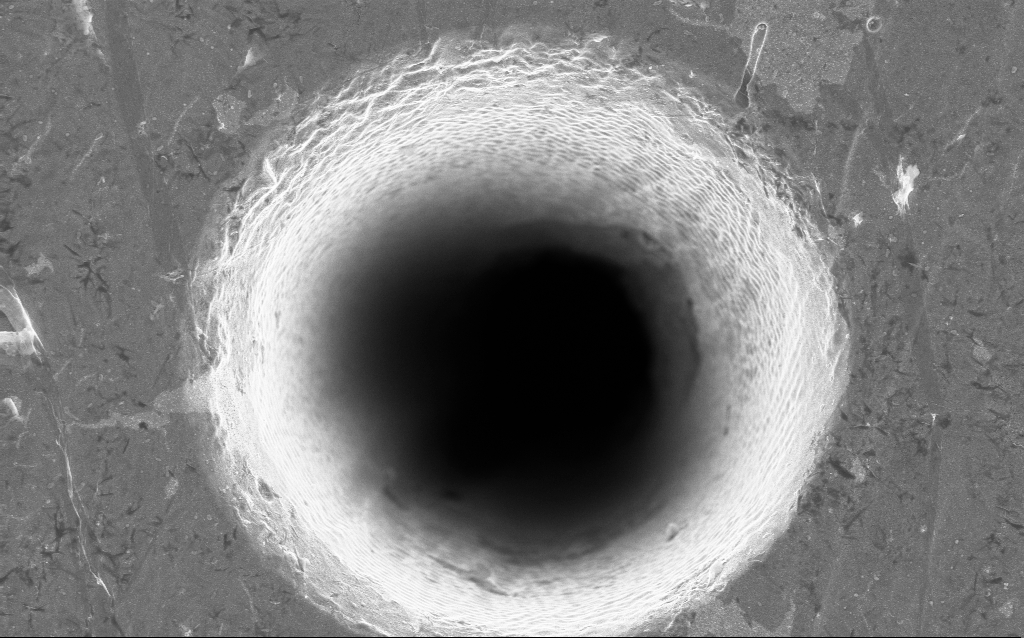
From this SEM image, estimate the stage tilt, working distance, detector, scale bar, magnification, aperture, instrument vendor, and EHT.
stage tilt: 0°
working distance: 4.4 mm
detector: InLens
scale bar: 2000 nm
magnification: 15.74 K X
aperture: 30 µm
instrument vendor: Zeiss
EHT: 5 kV